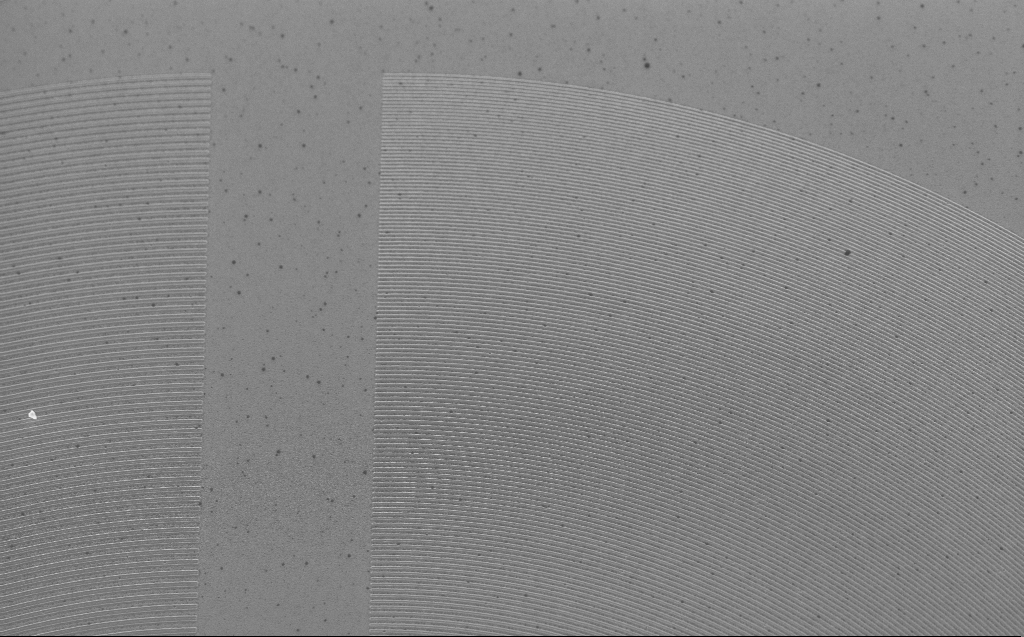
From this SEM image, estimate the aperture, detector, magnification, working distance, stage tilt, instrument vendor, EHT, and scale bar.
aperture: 30 µm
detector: InLens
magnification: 6.4 K X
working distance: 4 mm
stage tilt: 30°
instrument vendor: Zeiss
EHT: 5 kV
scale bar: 10000 nm